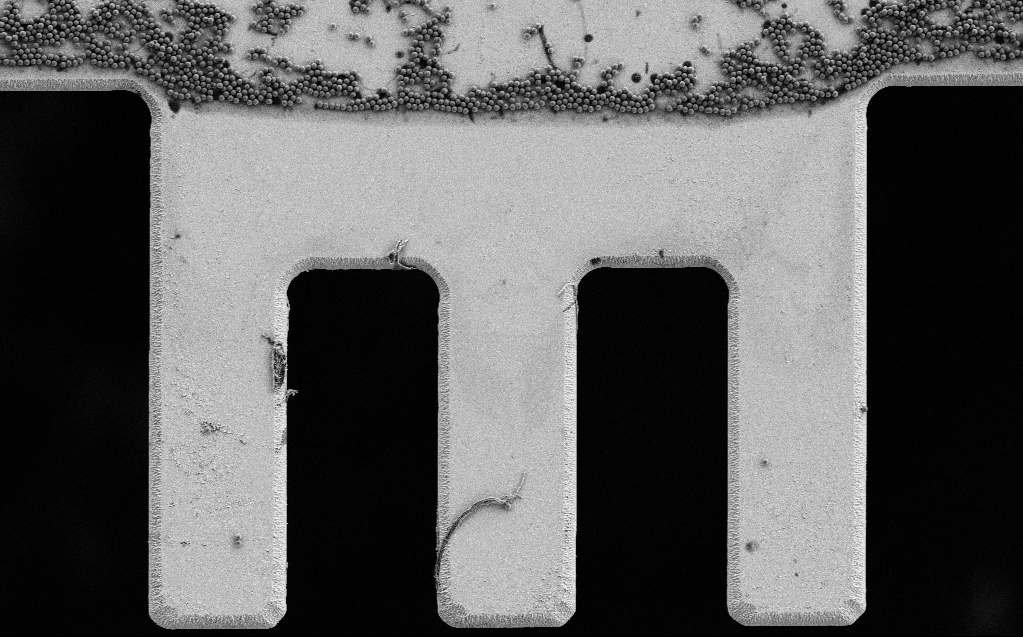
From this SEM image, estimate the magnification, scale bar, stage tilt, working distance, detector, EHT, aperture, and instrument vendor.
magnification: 2.66 K X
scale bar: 20000 nm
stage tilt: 0°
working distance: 7 mm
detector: SE2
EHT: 3 kV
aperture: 30 µm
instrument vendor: Zeiss